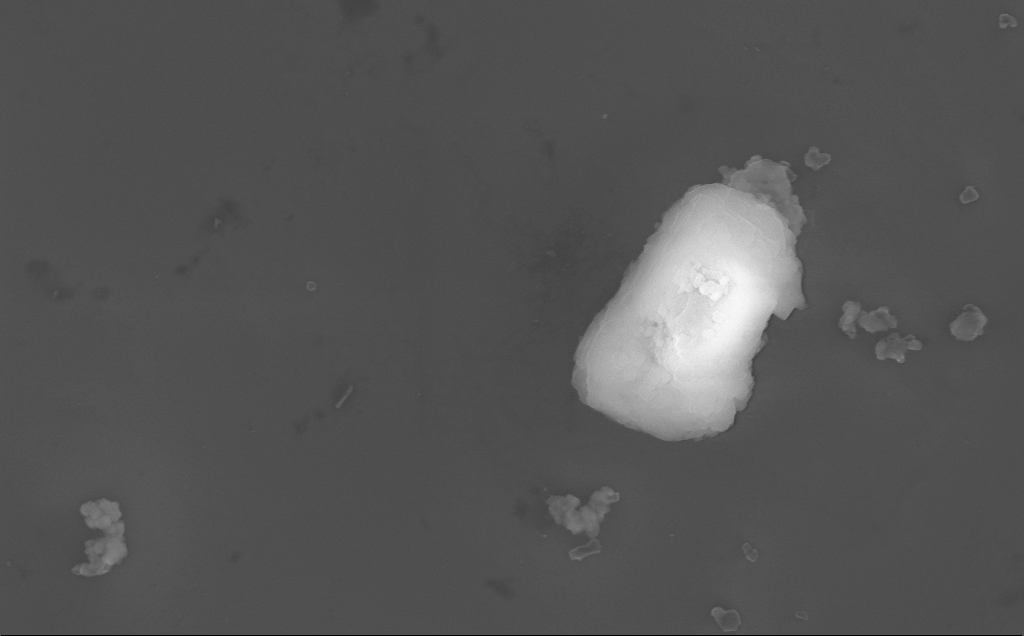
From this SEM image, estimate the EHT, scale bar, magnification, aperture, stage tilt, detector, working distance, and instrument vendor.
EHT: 10 kV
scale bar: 1000 nm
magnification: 37.18 K X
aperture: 30 µm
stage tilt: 0°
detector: InLens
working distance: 4 mm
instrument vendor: Zeiss